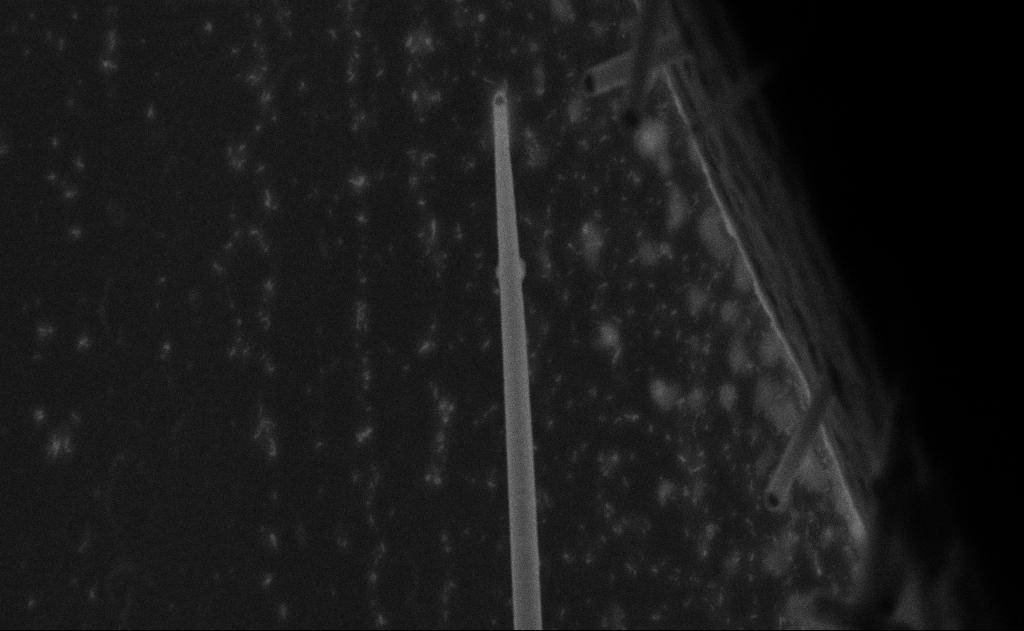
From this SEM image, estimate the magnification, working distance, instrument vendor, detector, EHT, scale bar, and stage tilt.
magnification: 114.23 K X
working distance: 9 mm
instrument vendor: Zeiss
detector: SE2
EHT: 20 kV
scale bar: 200 nm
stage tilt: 0°